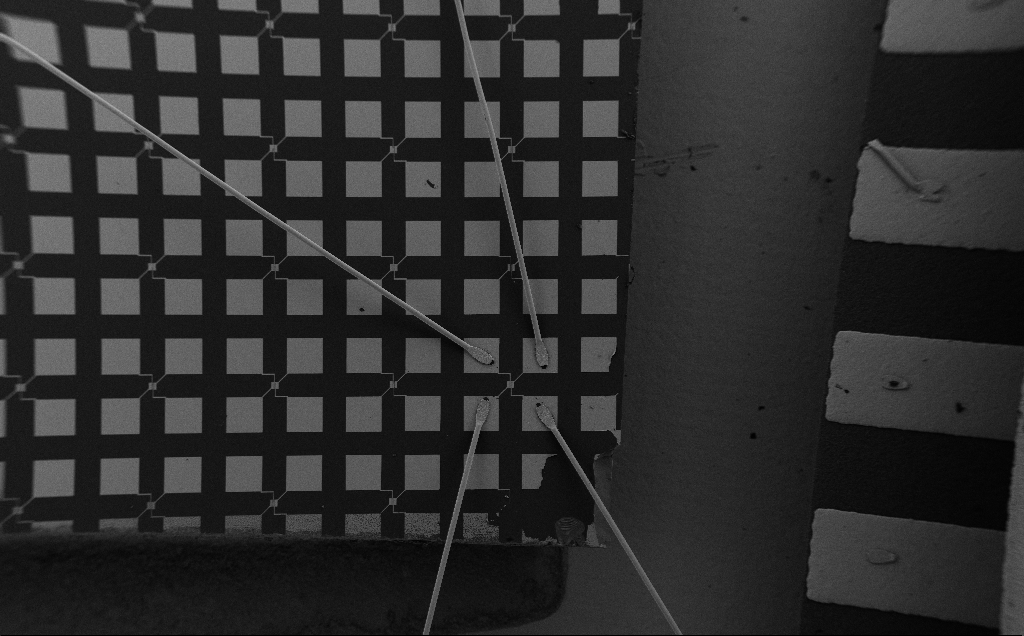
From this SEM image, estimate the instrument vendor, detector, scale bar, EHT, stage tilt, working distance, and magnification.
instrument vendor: Zeiss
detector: SE2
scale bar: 200000 nm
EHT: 5 kV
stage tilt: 0°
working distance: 6 mm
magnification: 0.087 K X